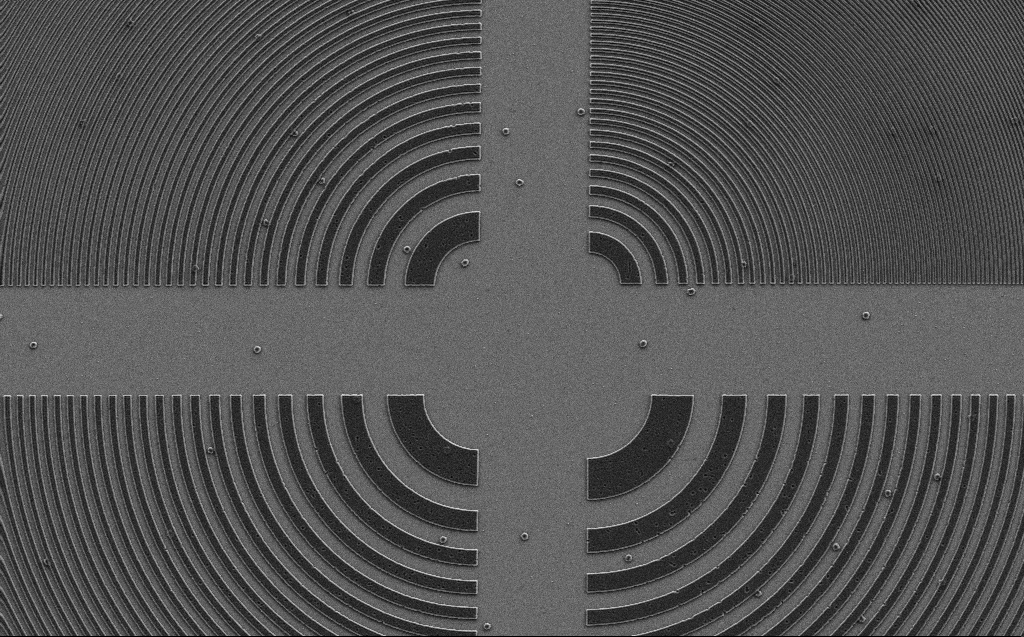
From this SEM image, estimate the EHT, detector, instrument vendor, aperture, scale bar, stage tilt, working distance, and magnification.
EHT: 3 kV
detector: SE2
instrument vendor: Zeiss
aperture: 30 µm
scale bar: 10000 nm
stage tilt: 0°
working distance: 6 mm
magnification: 4.04 K X